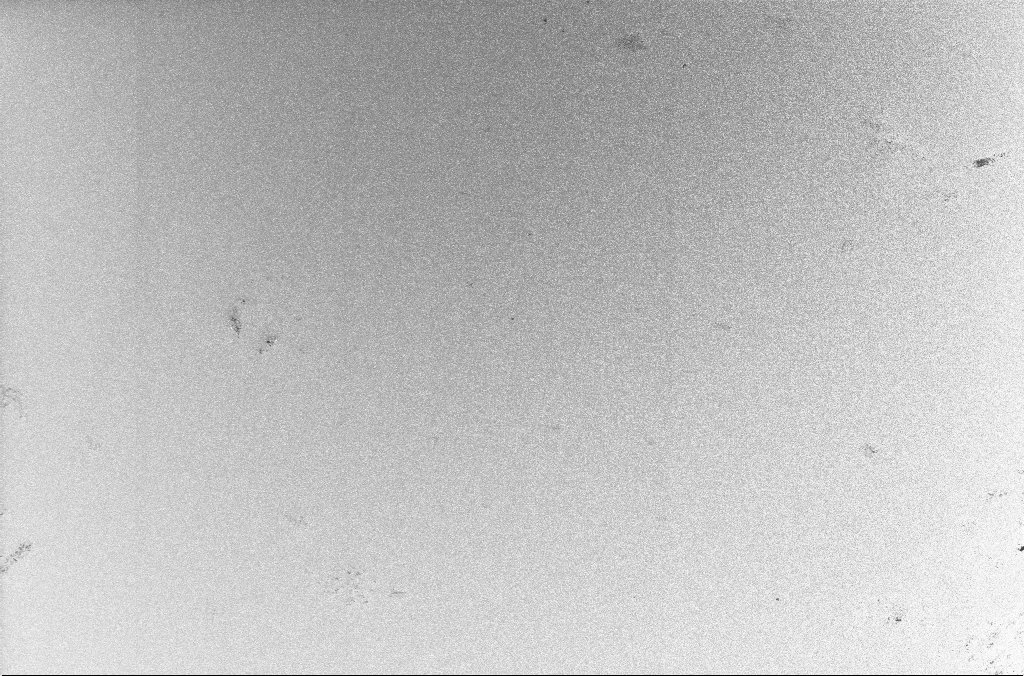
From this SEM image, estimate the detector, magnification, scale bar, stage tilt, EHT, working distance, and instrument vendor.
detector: InLens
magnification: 1 K X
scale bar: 20000 nm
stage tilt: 0°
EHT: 5 kV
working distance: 1.9 mm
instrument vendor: Zeiss